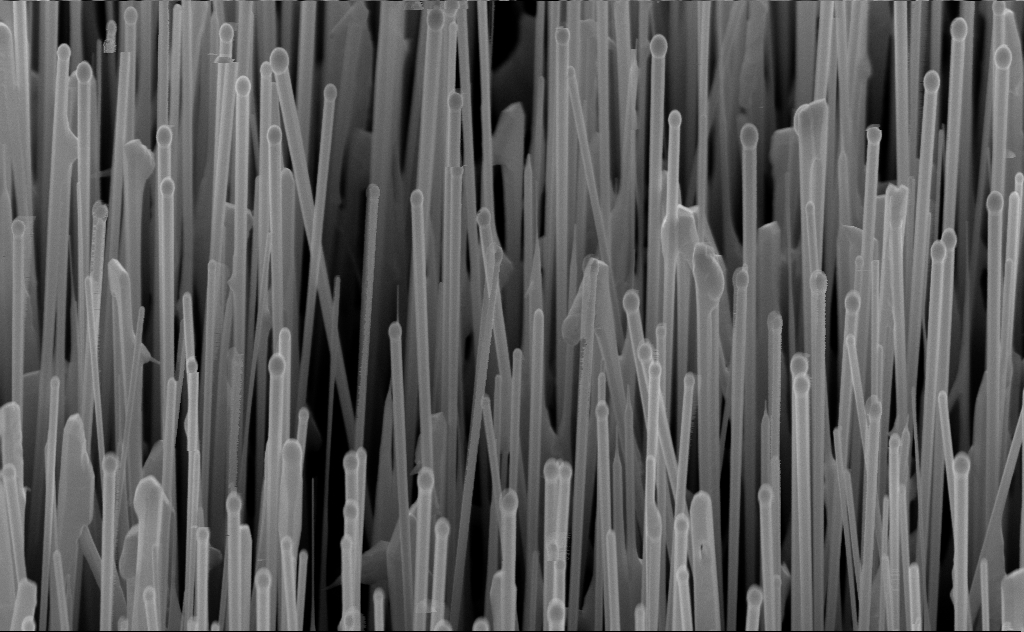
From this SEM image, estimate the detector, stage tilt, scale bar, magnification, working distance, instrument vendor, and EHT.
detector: InLens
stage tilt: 45°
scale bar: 1000 nm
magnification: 40 K X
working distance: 6 mm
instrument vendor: Zeiss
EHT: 10 kV